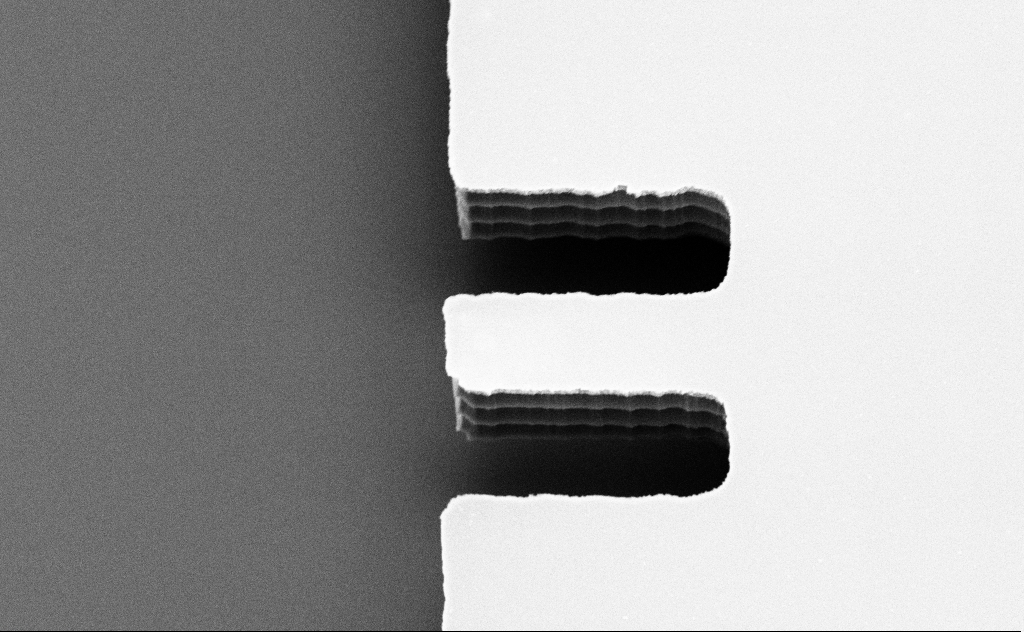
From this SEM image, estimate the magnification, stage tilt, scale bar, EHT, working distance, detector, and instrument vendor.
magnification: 7.12 K X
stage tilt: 43°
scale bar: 10000 nm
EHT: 5 kV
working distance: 10 mm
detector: SE2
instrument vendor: Zeiss